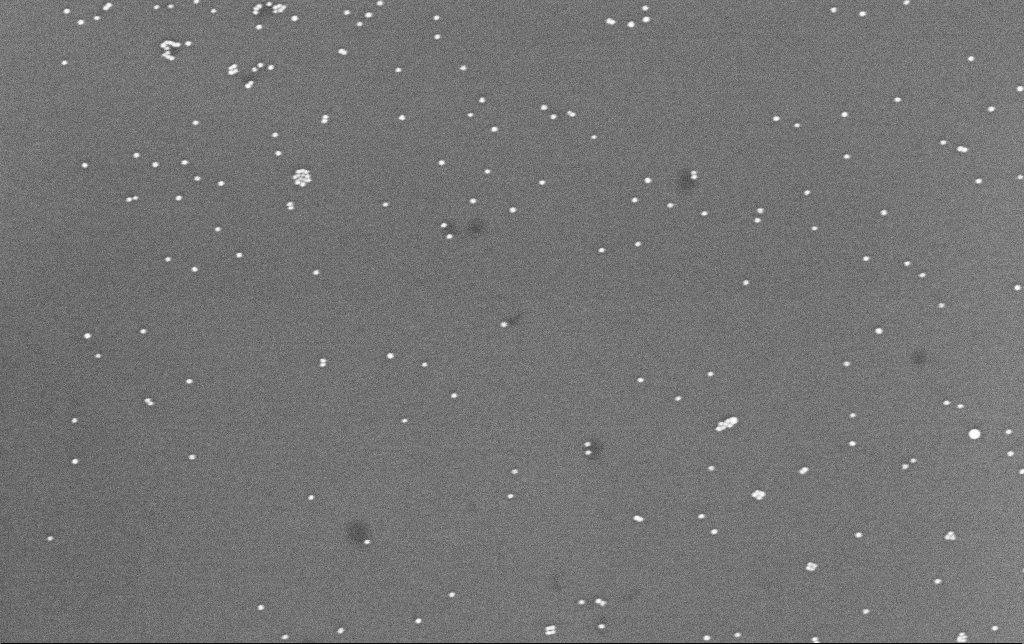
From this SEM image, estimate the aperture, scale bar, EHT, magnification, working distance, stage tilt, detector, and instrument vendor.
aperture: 30 µm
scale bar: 200 nm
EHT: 8 kV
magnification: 100 K X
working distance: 6.6 mm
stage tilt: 0°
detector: InLens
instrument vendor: Zeiss